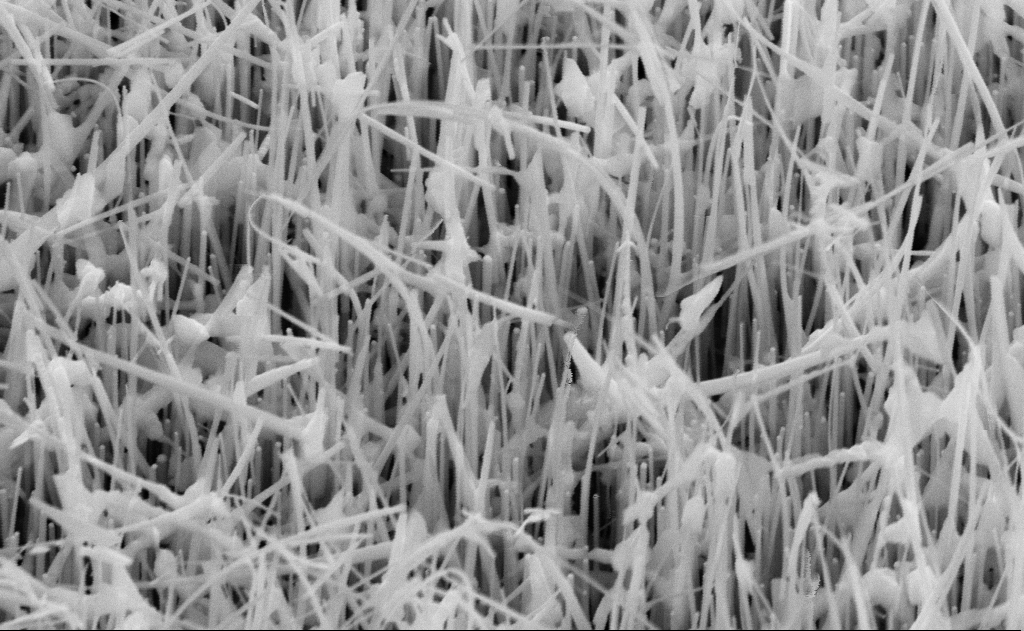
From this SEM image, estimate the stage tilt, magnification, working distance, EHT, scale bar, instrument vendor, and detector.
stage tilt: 45°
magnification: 60 K X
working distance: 14 mm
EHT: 10 kV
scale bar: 1000 nm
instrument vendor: Zeiss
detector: SE2